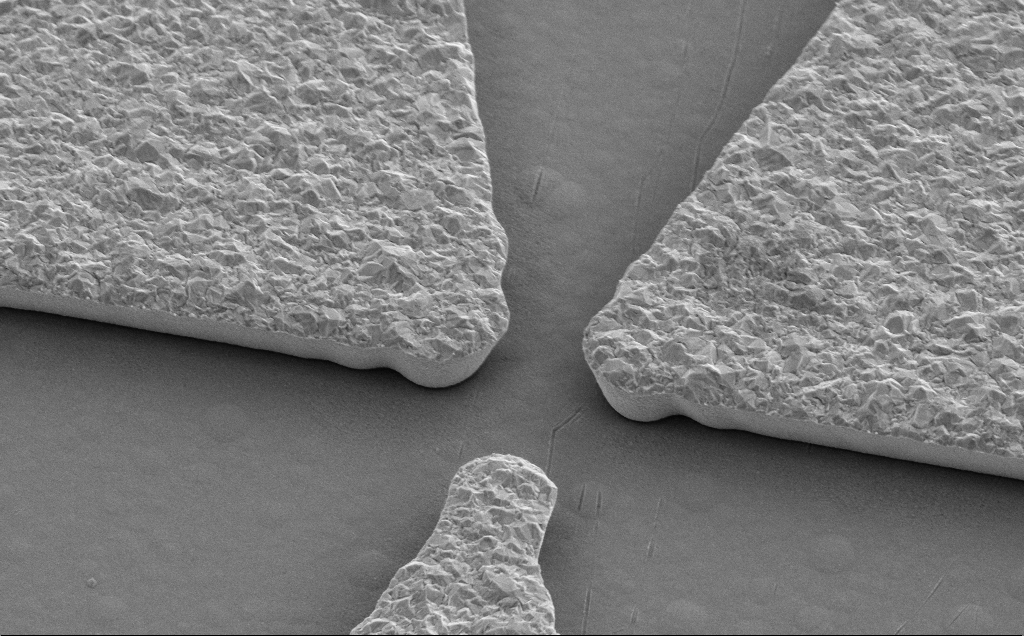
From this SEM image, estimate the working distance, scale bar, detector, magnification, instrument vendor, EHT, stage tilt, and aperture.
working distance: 13 mm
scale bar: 2000 nm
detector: SE2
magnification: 15.93 K X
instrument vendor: Zeiss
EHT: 5 kV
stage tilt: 35°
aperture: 30 µm